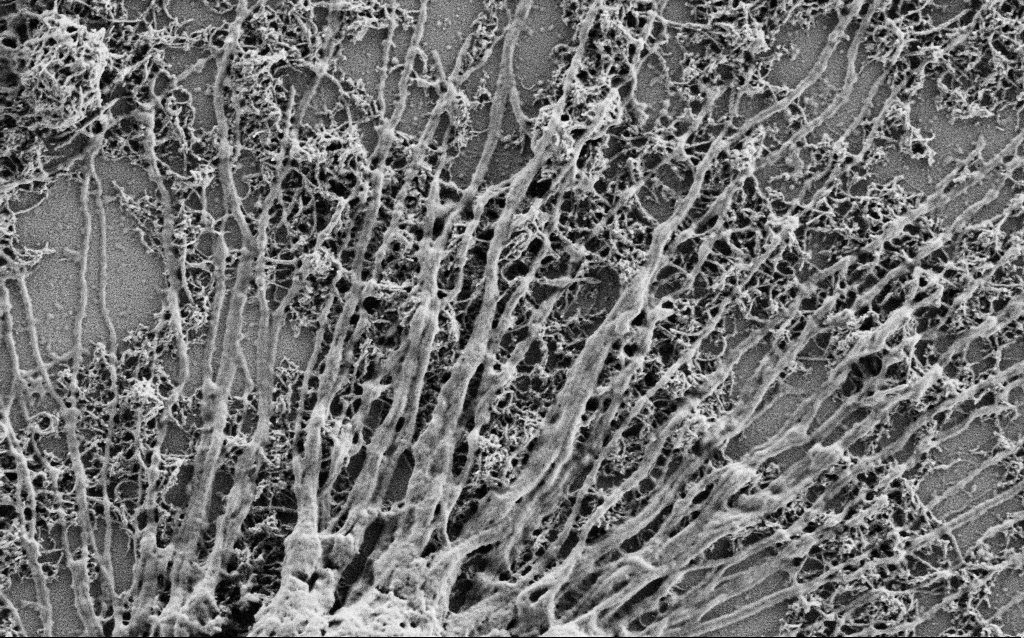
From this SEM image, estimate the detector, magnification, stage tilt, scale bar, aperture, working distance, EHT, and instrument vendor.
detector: SE2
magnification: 25 K X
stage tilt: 0°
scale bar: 2000 nm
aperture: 30 µm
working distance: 4 mm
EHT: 1 kV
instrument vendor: Zeiss